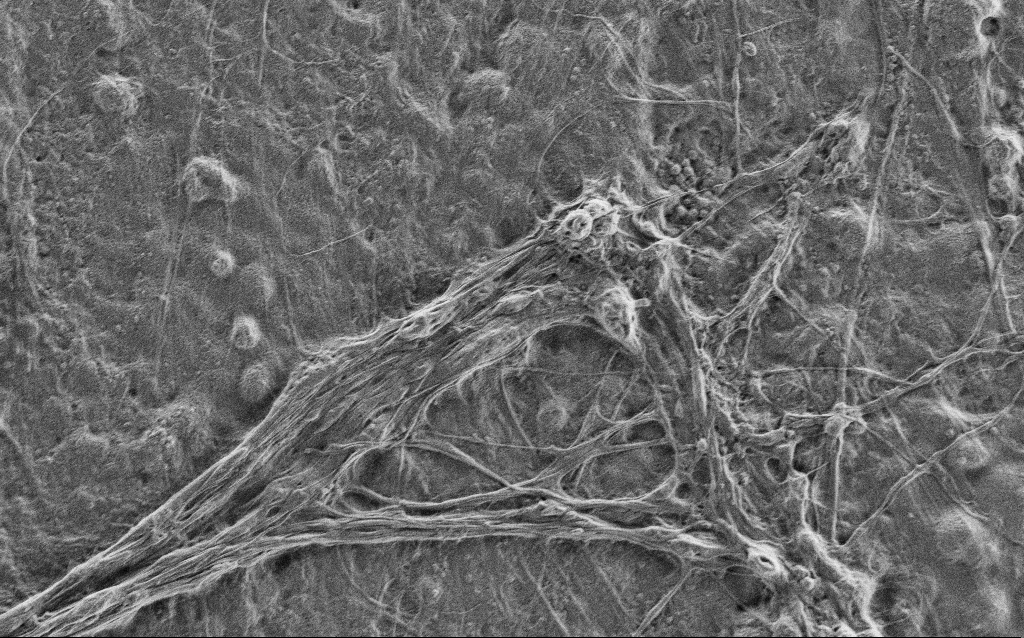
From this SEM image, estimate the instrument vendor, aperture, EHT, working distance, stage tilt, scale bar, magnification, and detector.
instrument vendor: Zeiss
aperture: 30 µm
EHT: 0.9 kV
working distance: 4 mm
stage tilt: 0°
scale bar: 10000 nm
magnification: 5 K X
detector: SE2